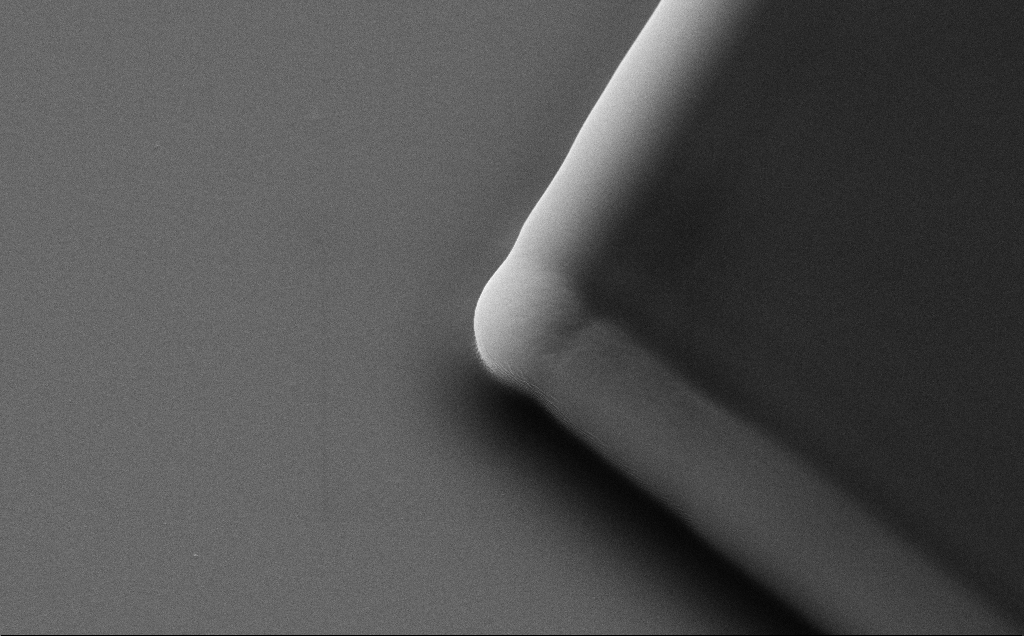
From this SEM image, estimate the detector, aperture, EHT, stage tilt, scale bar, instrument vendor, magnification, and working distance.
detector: SE2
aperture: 30 µm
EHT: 10 kV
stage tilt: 35°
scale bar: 1000 nm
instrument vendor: Zeiss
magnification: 25.06 K X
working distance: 8 mm